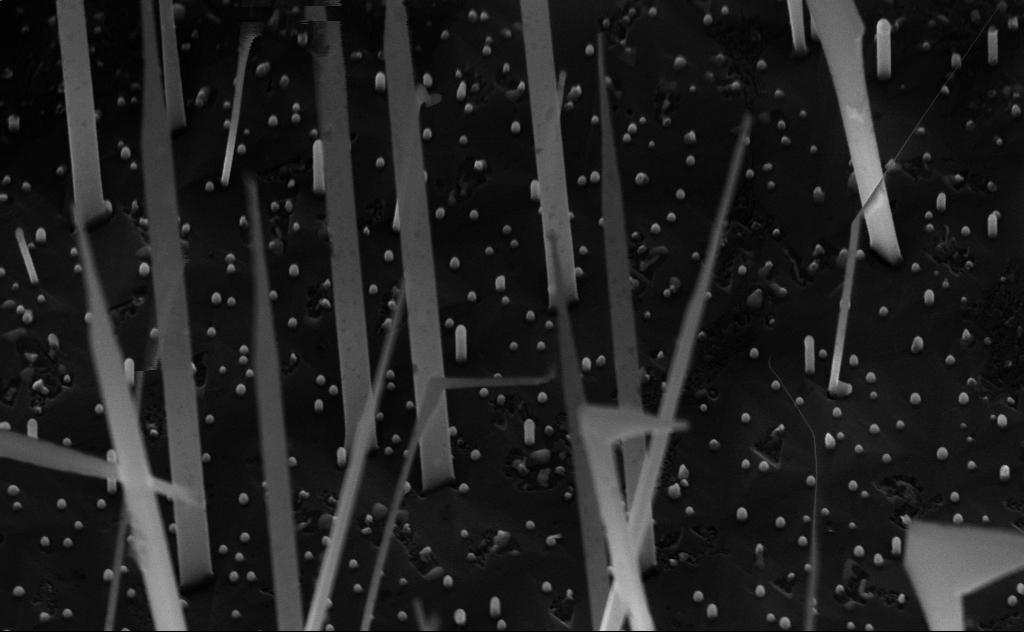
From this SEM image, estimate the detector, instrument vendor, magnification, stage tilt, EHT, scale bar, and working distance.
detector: InLens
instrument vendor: Zeiss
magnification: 80 K X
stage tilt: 45°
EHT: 10 kV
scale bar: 200 nm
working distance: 6 mm